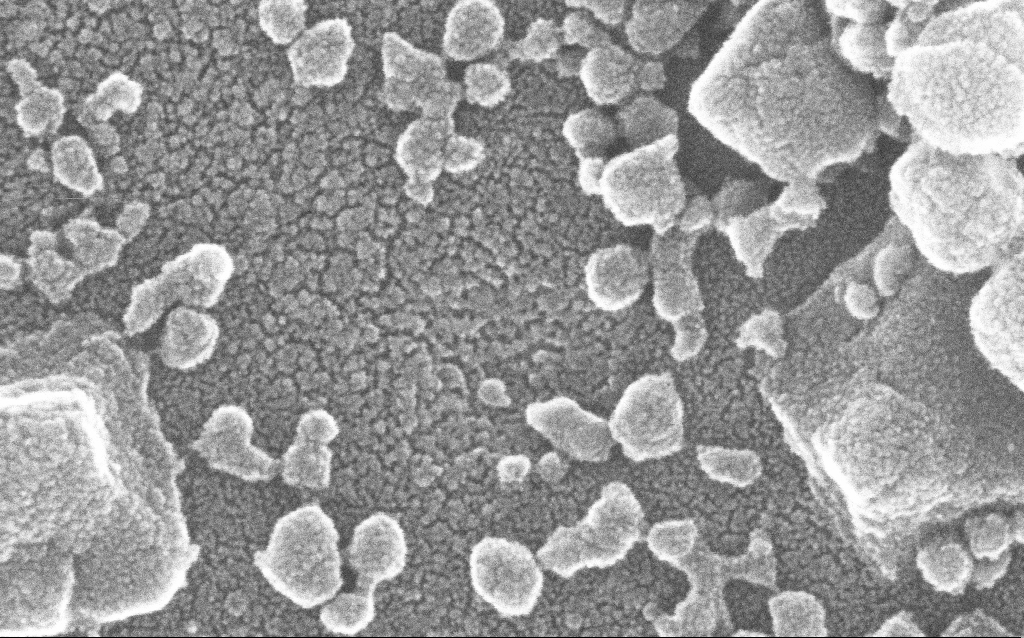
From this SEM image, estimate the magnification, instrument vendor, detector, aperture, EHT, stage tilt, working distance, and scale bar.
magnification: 500 K X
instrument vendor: Zeiss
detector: InLens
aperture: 30 µm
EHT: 20 kV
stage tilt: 0°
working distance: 1.9 mm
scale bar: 100 nm